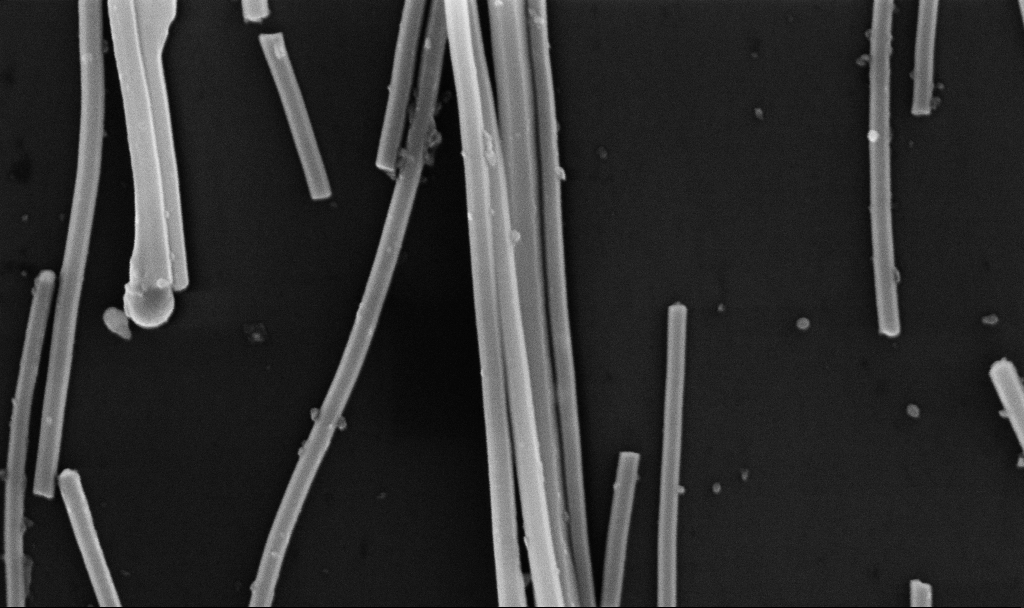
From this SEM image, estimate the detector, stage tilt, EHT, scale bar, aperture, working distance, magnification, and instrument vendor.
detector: InLens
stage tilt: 0°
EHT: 10 kV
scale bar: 200 nm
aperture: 30 µm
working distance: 6.7 mm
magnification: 81.22 K X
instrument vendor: Zeiss